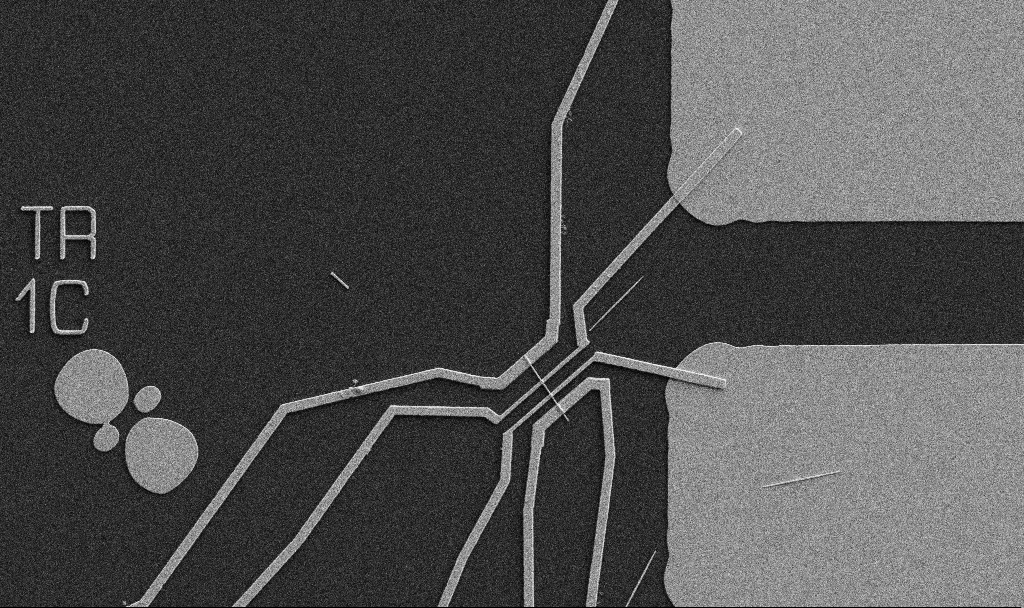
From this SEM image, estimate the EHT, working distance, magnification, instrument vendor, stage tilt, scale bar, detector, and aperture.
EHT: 5 kV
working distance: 10.7 mm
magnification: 5 K X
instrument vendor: Zeiss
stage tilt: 0°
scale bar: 10000 nm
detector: SE2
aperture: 30 µm